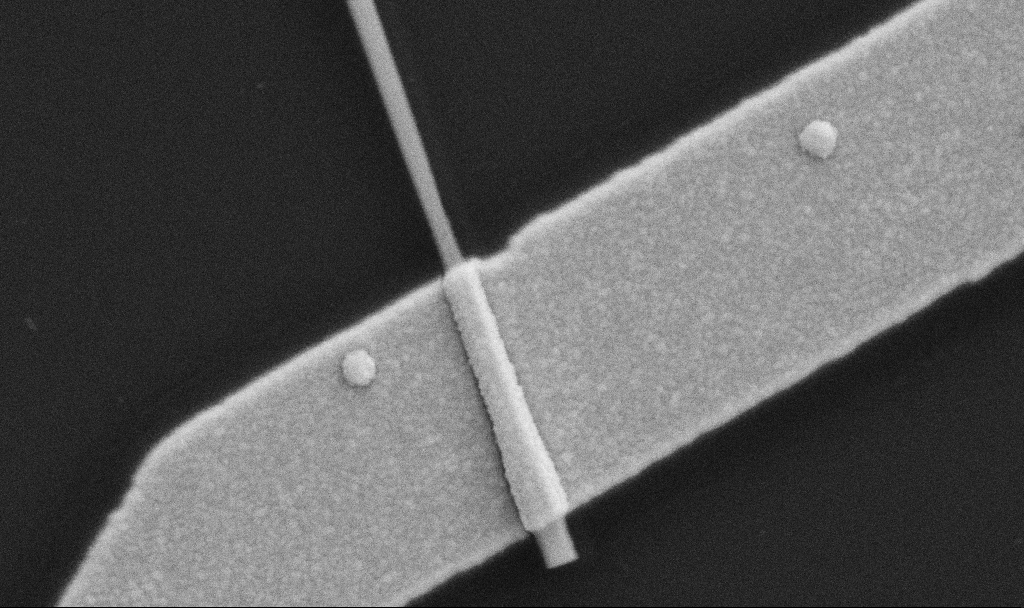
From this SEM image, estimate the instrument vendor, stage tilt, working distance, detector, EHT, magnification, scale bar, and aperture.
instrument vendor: Zeiss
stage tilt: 0°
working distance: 10.7 mm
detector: SE2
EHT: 5 kV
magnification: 100 K X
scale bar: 200 nm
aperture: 30 µm